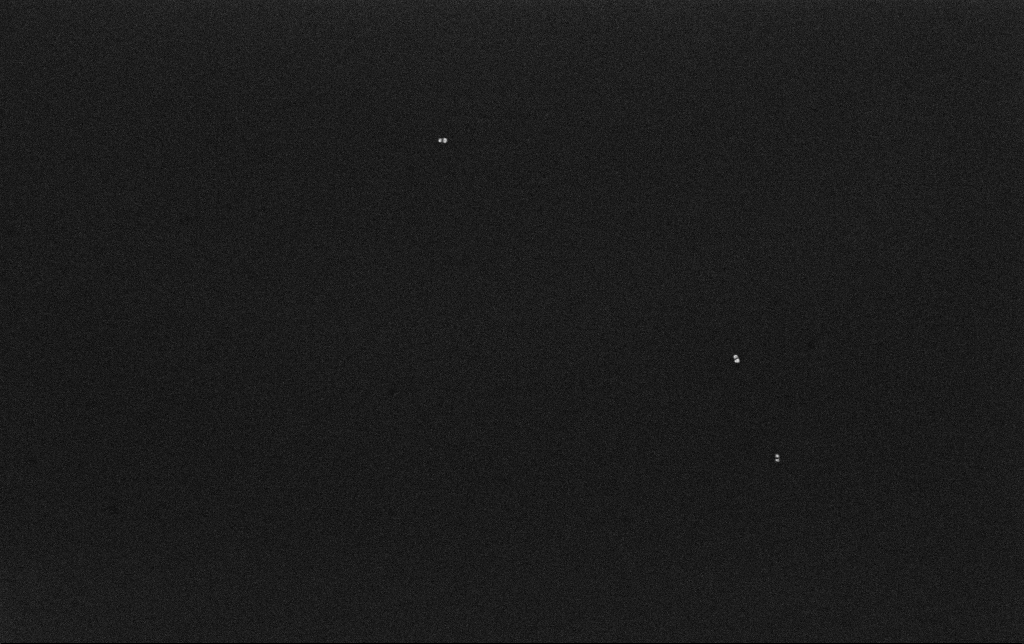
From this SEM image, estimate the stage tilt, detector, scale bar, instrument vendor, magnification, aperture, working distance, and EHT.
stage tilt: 0°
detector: InLens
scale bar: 200 nm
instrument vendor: Zeiss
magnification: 100 K X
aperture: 30 µm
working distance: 3.2 mm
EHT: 10 kV